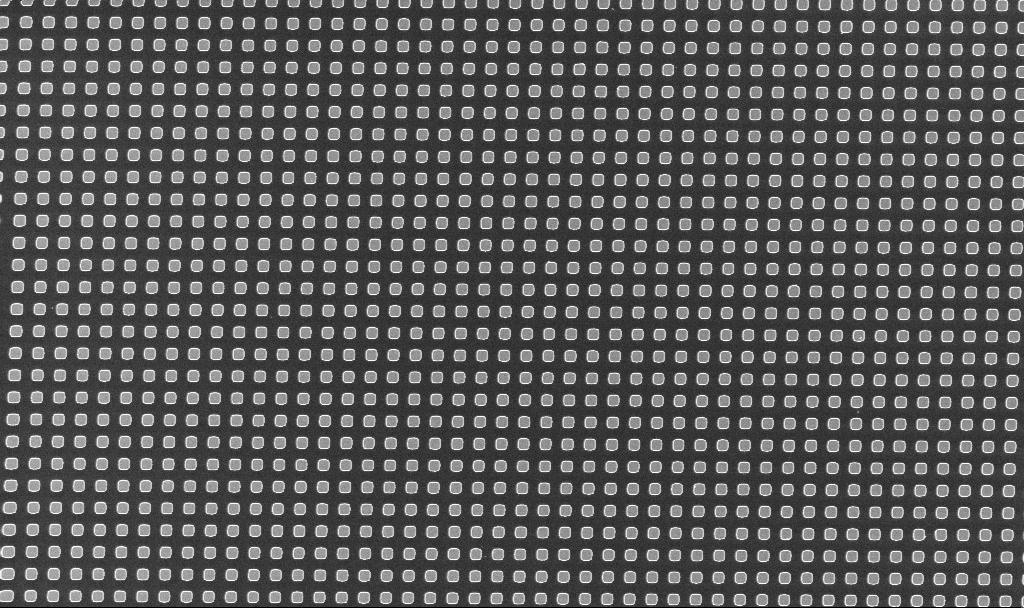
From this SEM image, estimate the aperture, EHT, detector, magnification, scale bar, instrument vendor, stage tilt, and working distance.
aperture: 30 µm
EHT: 3 kV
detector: InLens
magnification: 2.05 K X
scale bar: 10000 nm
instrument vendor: Zeiss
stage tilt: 0°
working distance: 3.3 mm